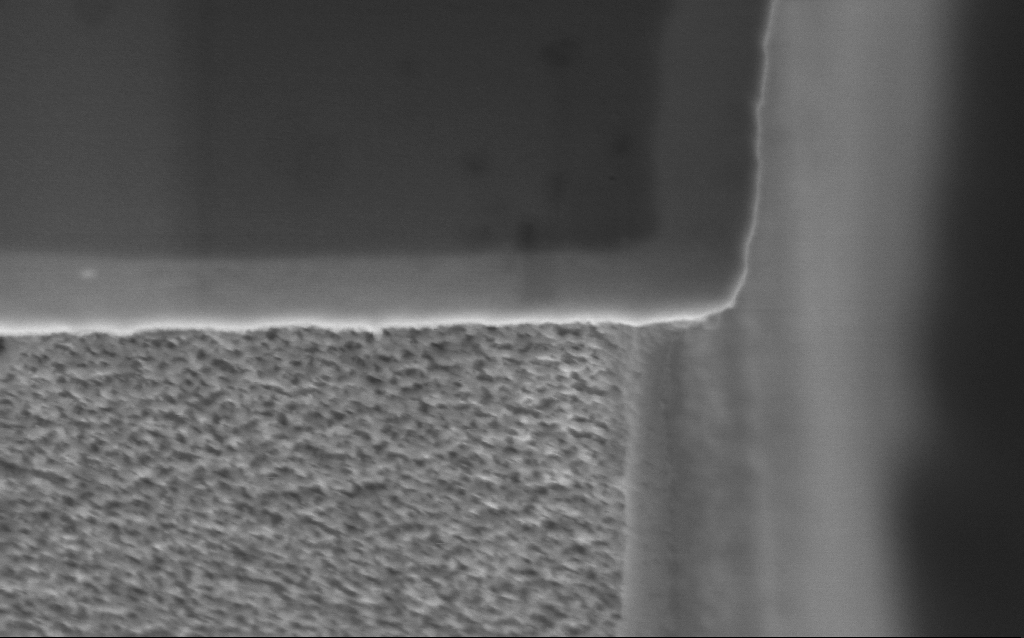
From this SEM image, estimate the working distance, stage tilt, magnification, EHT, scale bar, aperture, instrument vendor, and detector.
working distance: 6.2 mm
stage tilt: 45°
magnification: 101.28 K X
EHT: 3 kV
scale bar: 200 nm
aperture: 30 µm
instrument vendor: Zeiss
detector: InLens